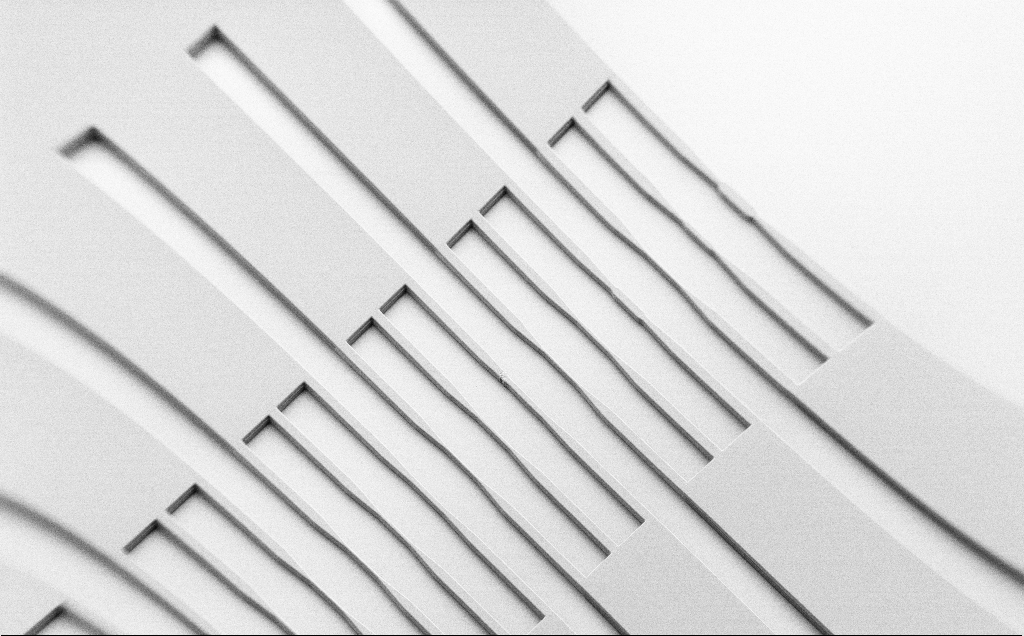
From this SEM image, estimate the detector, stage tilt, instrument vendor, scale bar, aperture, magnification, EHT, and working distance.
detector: SE2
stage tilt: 45°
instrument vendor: Zeiss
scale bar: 100000 nm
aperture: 30 µm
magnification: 0.159 K X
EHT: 1.3 kV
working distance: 8 mm